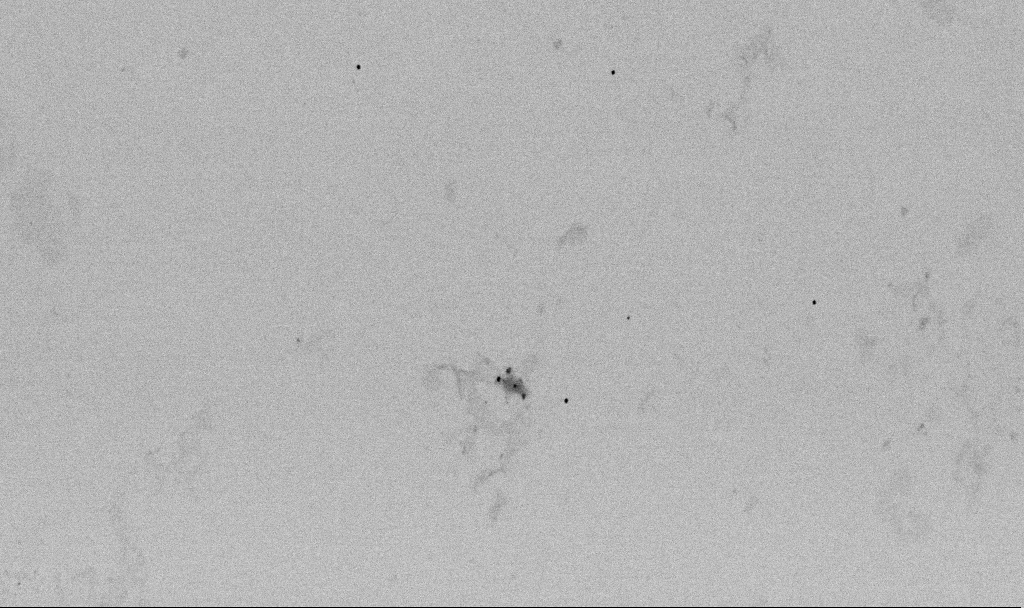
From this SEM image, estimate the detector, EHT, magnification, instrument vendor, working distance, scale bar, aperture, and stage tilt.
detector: SE2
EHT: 2 kV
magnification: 50 K X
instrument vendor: Zeiss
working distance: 6.5 mm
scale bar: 1000 nm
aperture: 30 µm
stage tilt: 0°